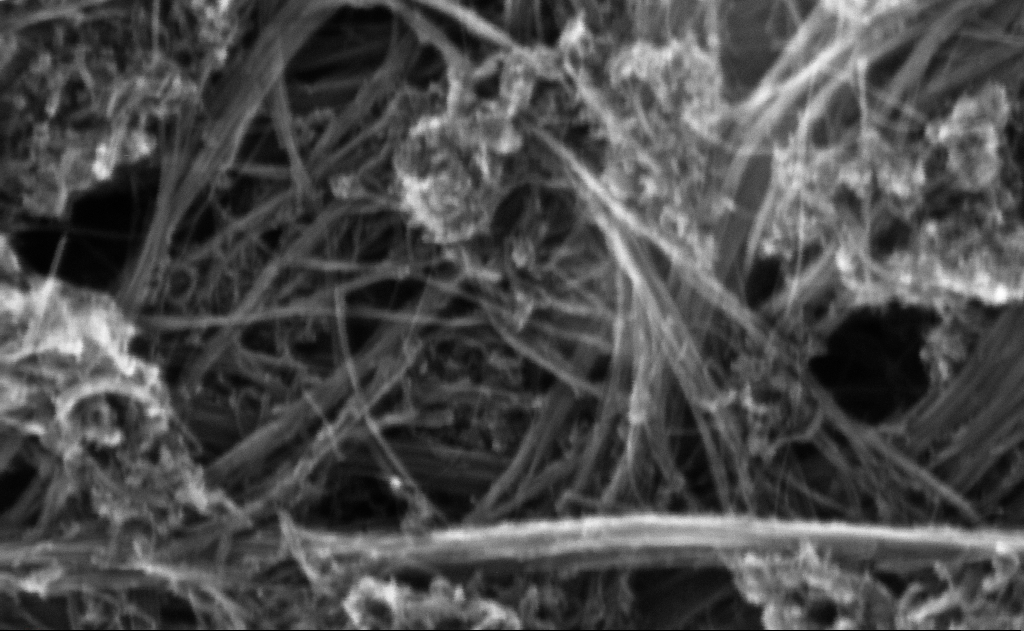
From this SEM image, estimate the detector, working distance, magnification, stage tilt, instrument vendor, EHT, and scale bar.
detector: InLens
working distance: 6 mm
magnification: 388.65 K X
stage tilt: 0°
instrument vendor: Zeiss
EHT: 10 kV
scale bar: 100 nm